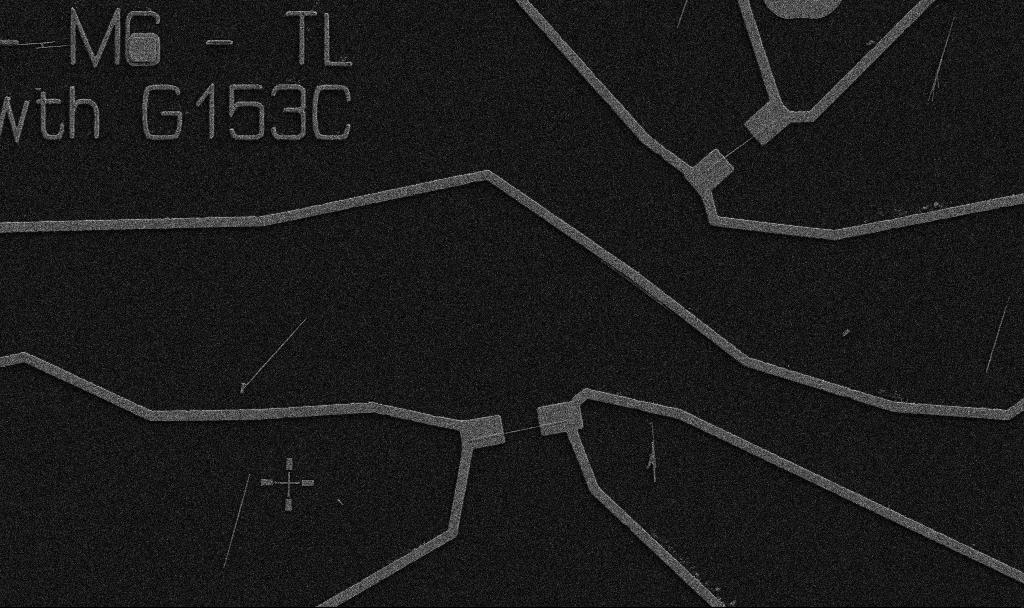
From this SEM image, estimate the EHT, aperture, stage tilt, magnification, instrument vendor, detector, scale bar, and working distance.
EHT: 5 kV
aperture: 30 µm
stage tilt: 0°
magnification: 5 K X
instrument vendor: Zeiss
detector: SE2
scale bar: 10000 nm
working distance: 10.7 mm